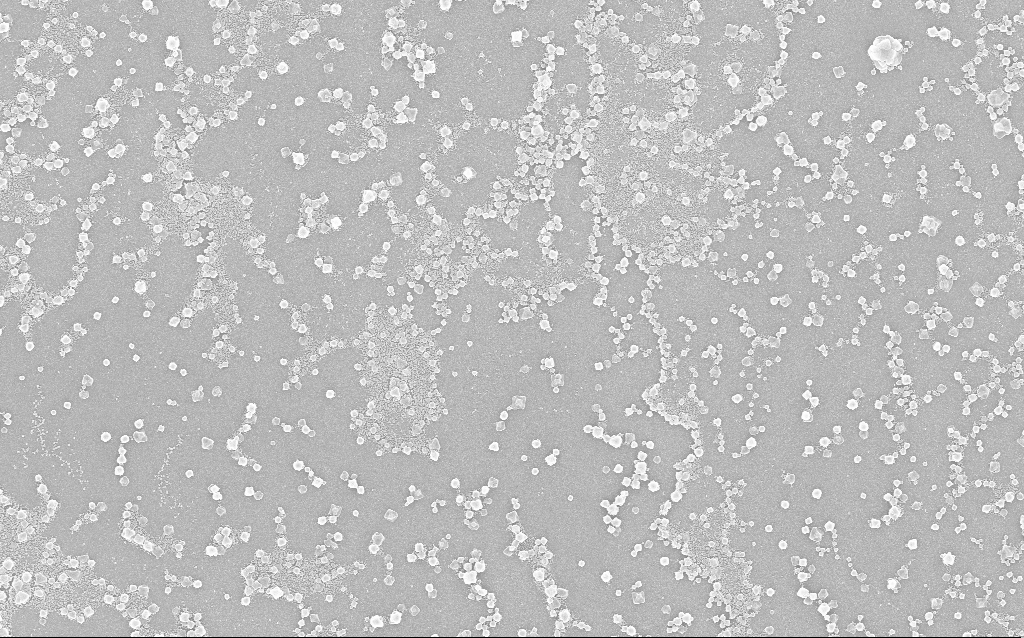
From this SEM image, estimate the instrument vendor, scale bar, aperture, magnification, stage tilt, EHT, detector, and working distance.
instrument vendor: Zeiss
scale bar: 2000 nm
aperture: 30 µm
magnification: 10 K X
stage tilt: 0°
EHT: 20 kV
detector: InLens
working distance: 1.8 mm